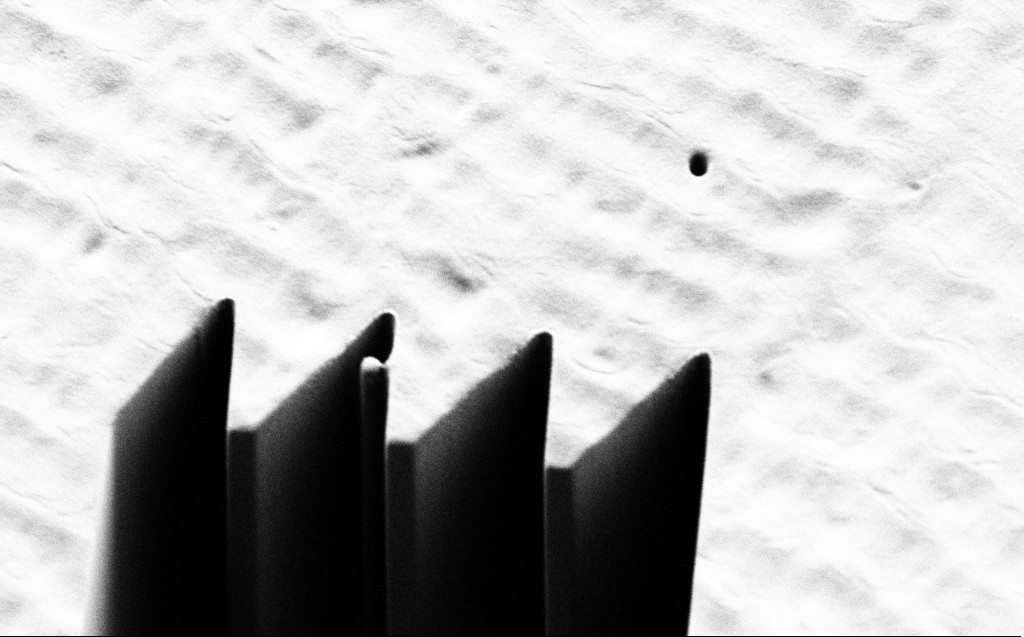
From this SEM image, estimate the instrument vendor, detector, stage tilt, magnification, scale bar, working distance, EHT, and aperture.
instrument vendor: Zeiss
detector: SE2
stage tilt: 44.9°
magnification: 5.14 K X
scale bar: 10000 nm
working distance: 7 mm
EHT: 1 kV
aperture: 30 µm